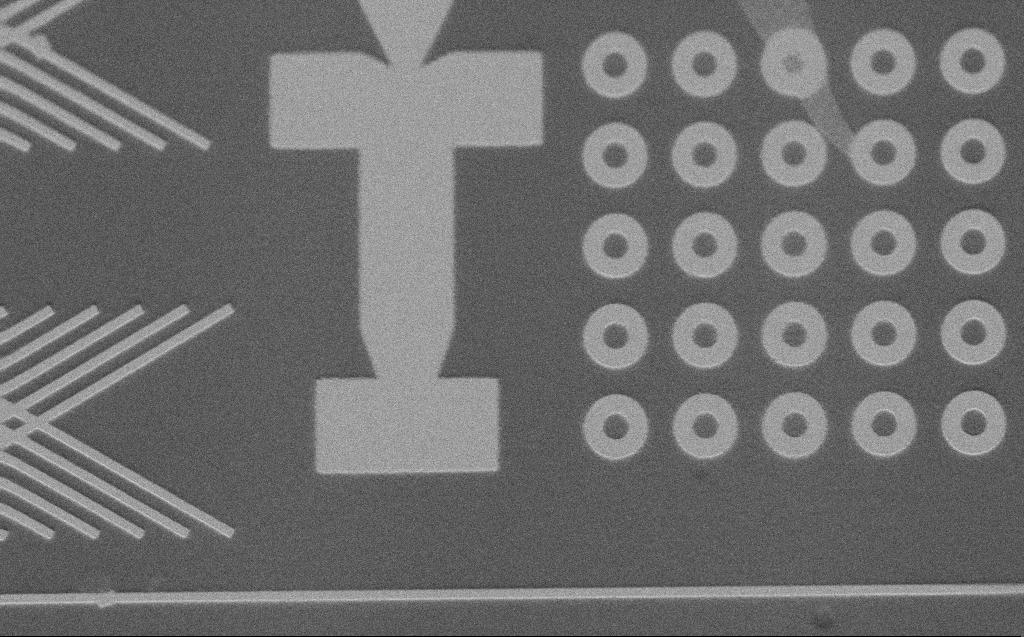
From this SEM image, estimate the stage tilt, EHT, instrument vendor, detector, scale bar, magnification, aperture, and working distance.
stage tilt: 45°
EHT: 3 kV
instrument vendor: Zeiss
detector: SE2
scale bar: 10000 nm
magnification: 3.32 K X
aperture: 30 µm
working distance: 5 mm